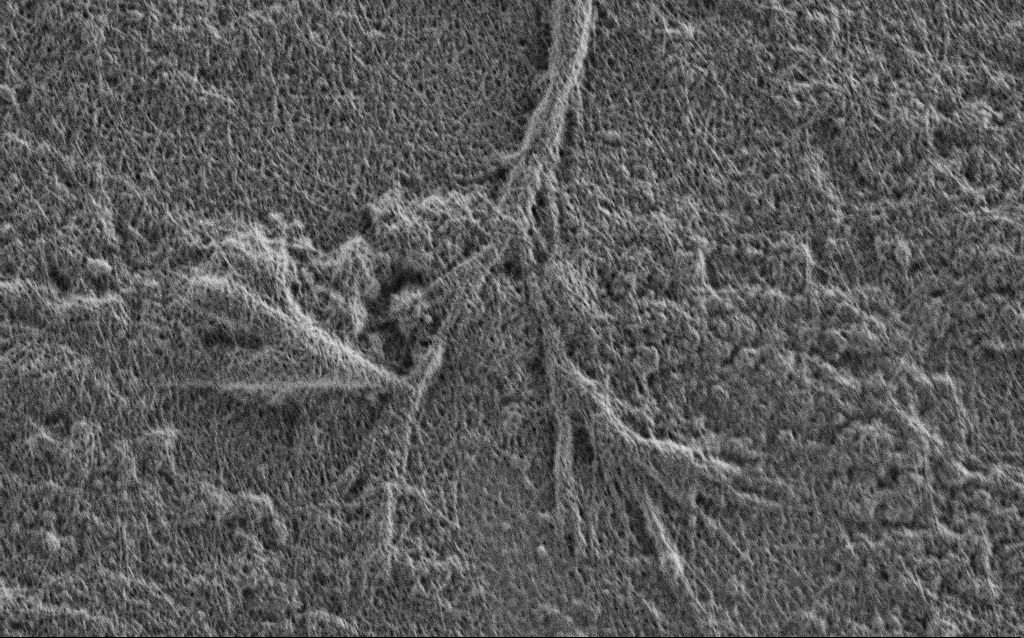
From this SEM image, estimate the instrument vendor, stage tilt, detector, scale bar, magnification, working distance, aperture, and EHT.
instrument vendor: Zeiss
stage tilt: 0°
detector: SE2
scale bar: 2000 nm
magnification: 15 K X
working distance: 4 mm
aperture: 30 µm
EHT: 1 kV